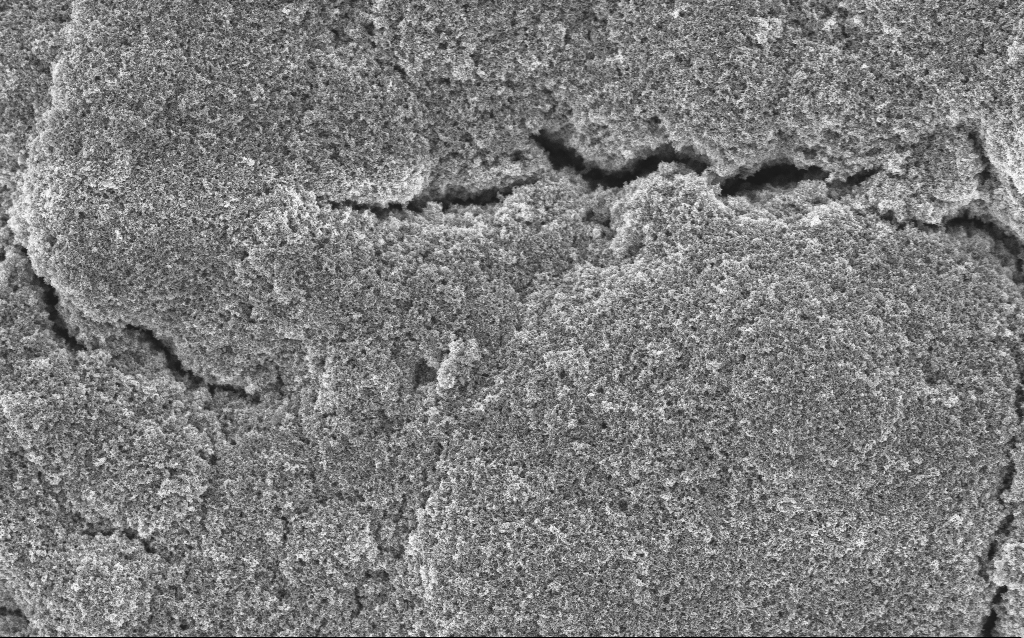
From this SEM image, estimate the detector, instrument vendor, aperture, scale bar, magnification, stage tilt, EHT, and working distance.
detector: InLens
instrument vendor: Zeiss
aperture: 30 µm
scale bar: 1000 nm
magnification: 15.33 K X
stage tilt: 0°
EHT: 5 kV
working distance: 4.2 mm